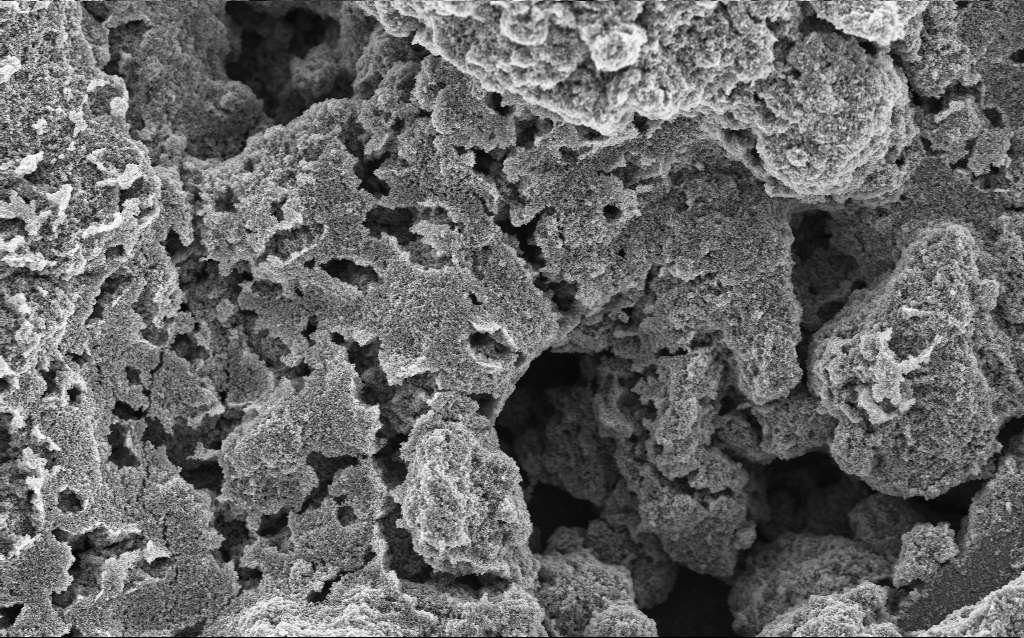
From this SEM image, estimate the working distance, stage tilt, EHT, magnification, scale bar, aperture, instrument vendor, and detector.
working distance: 4.4 mm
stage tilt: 0°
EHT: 5 kV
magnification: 6.42 K X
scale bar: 10000 nm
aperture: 30 µm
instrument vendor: Zeiss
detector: InLens